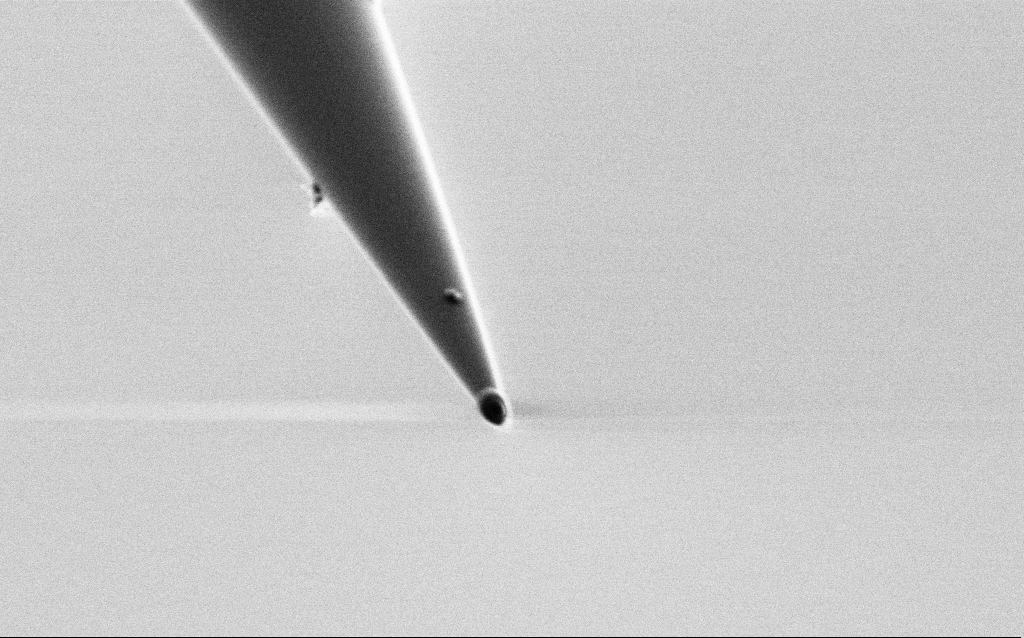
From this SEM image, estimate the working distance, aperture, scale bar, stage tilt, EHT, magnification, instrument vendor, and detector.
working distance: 6.6 mm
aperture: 30 µm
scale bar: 1000 nm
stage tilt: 45°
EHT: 1 kV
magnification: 50 K X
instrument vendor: Zeiss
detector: SE2